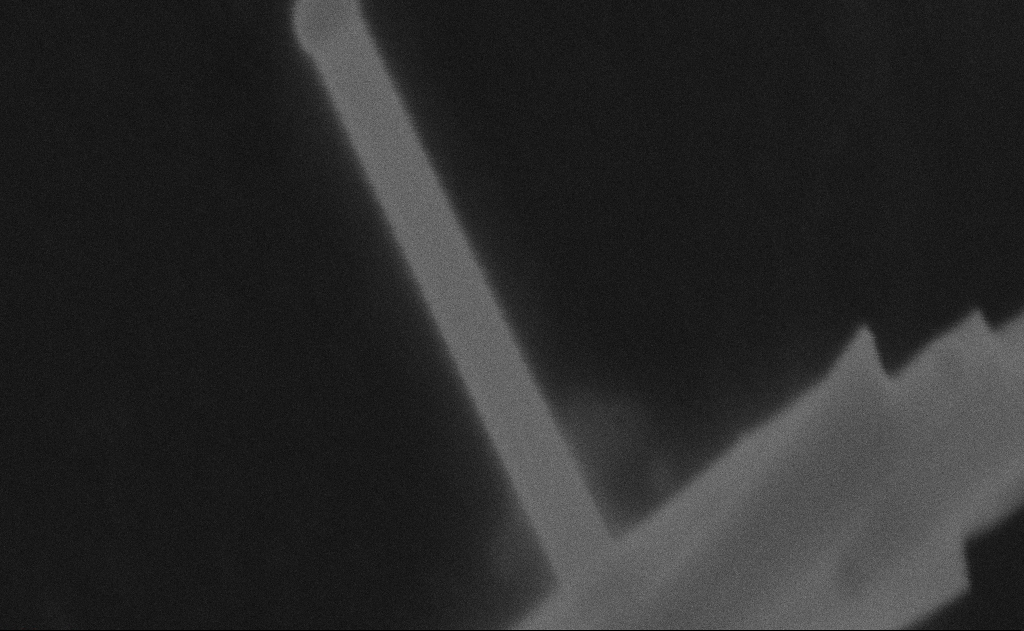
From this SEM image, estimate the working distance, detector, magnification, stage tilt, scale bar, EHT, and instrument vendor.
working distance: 11 mm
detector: SE2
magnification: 414.6 K X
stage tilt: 0°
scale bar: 100 nm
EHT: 20 kV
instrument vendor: Zeiss